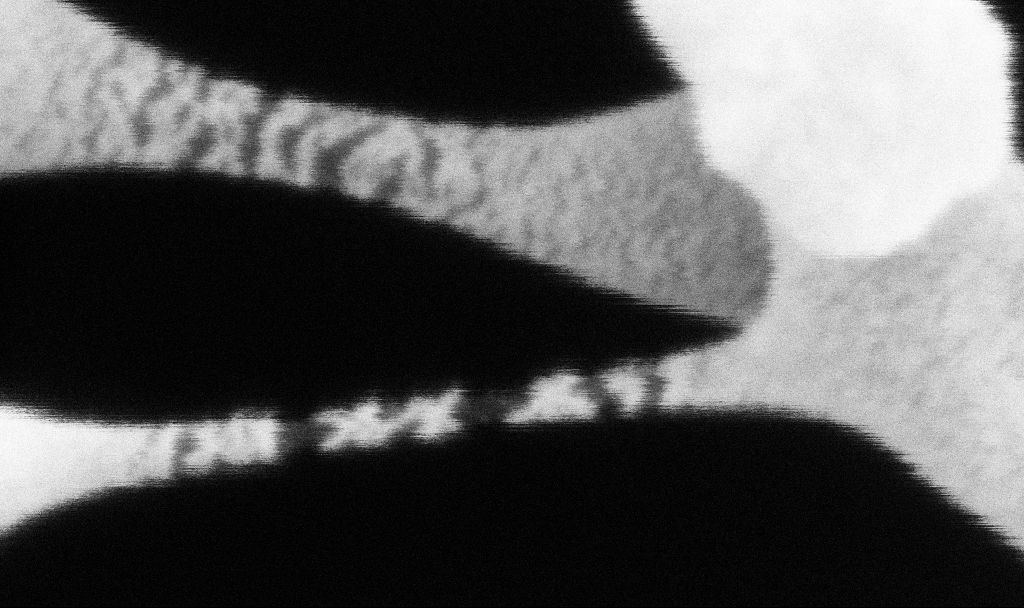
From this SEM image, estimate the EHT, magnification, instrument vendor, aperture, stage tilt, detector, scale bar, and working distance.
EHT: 2 kV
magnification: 800 K X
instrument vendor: Zeiss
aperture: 30 µm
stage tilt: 0°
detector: SE2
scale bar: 20 nm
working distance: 3.7 mm